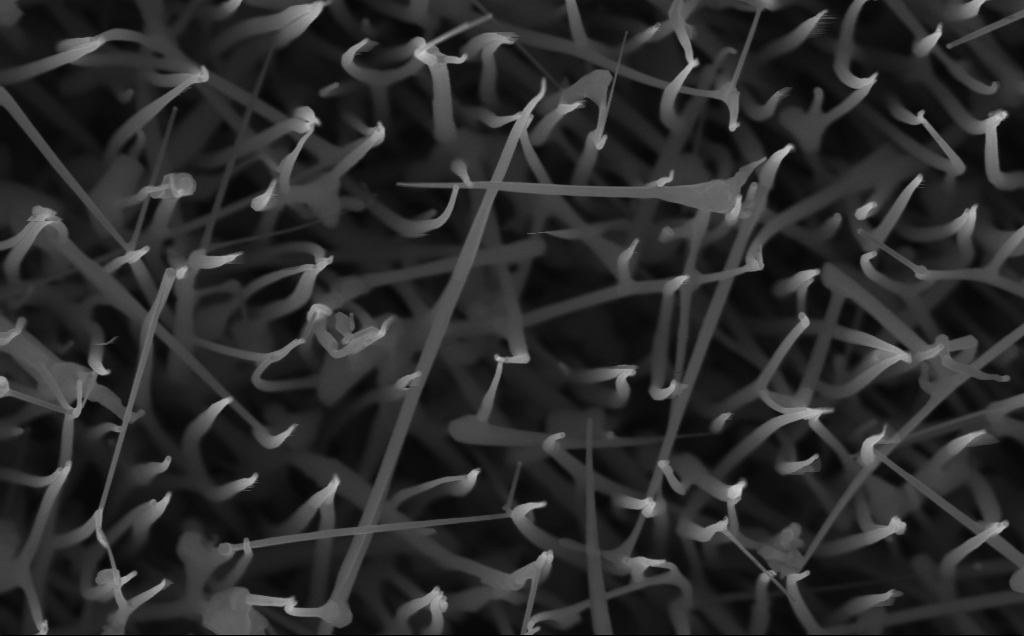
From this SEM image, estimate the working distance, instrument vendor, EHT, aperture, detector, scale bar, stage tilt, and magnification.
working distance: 4 mm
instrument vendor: Zeiss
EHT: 10 kV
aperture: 30 µm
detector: InLens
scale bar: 200 nm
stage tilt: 0°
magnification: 80 K X